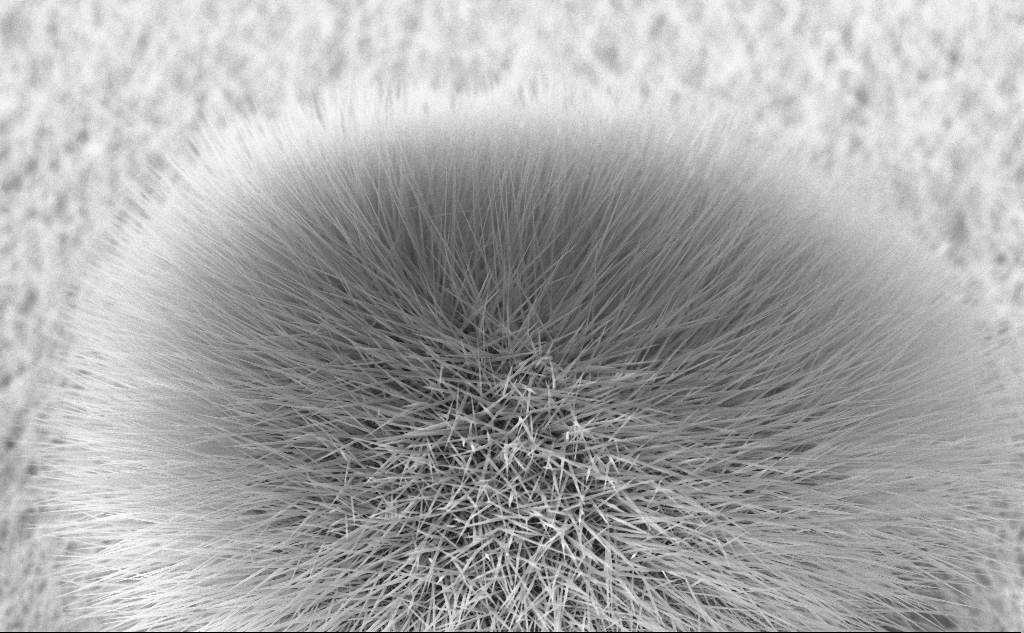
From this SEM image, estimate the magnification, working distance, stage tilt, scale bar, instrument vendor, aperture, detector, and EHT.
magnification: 9.93 K X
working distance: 4 mm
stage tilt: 45°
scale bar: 2000 nm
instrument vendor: Zeiss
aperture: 30 µm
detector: InLens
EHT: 10 kV